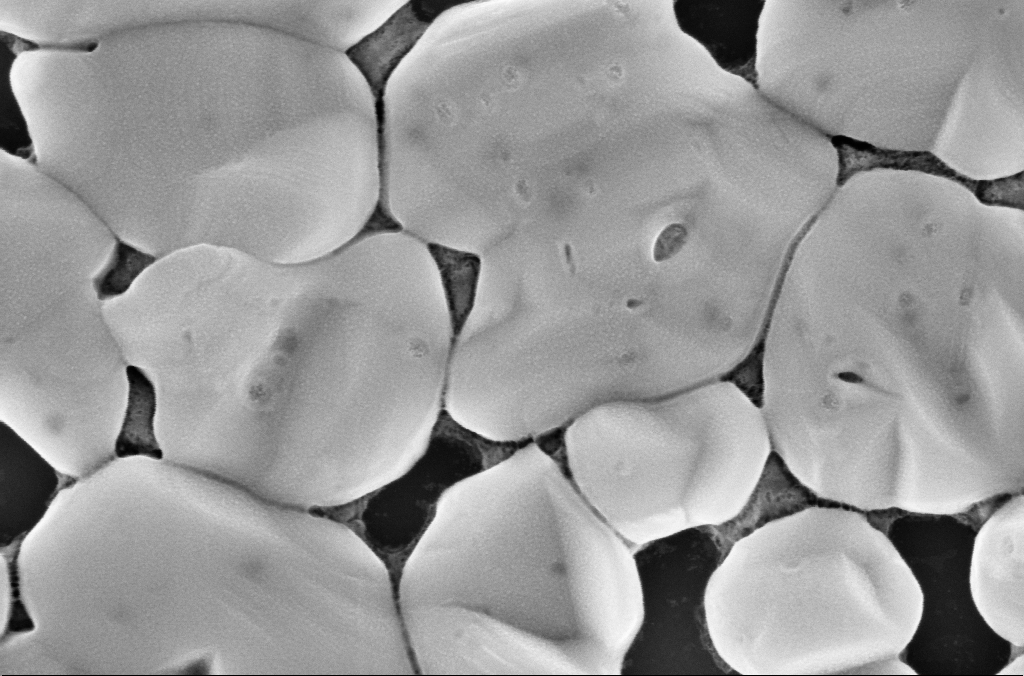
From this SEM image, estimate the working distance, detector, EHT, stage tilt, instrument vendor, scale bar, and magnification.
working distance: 3 mm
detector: InLens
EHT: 5 kV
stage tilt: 0°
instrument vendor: Zeiss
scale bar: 300 nm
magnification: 139.66 K X